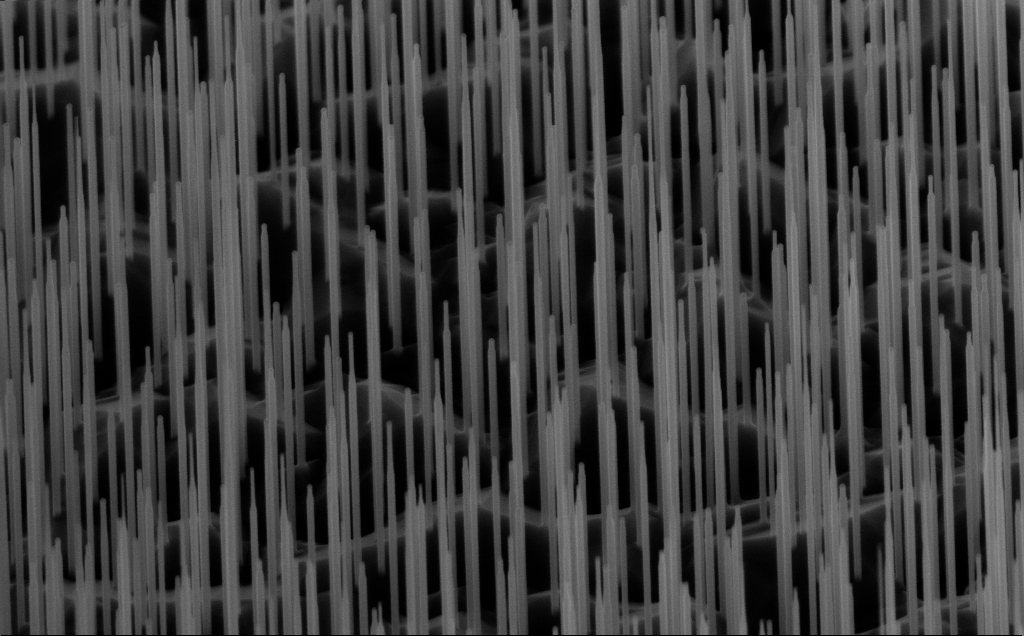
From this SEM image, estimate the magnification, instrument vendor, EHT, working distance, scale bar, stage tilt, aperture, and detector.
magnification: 40 K X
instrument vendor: Zeiss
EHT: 10 kV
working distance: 6 mm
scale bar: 1000 nm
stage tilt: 45°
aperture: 30 µm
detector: InLens